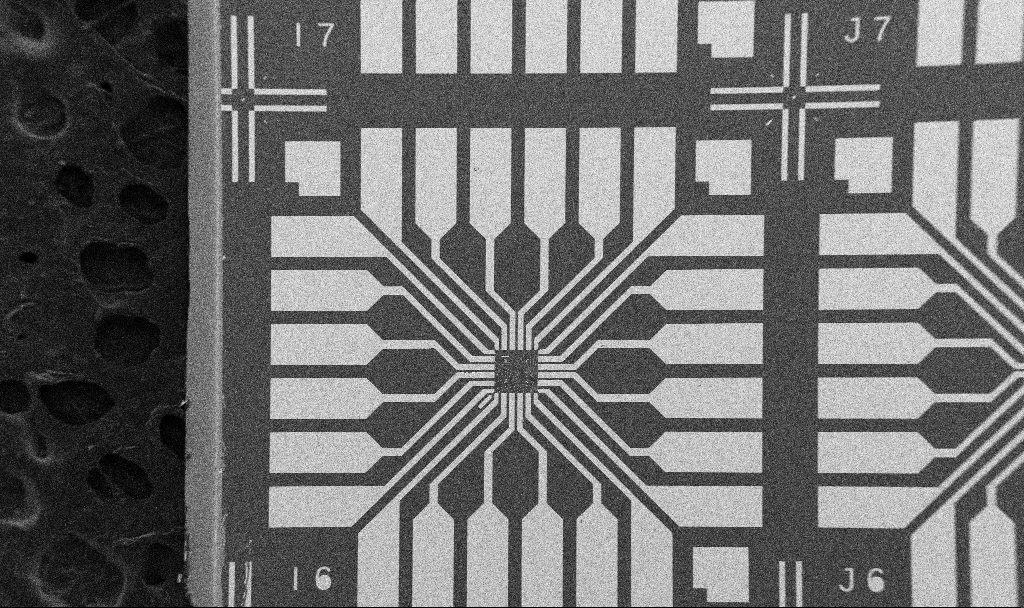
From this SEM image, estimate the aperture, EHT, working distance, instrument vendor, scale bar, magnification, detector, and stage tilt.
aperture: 30 µm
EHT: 5 kV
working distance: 10.7 mm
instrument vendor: Zeiss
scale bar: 200000 nm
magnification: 0.1 K X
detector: SE2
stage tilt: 0°